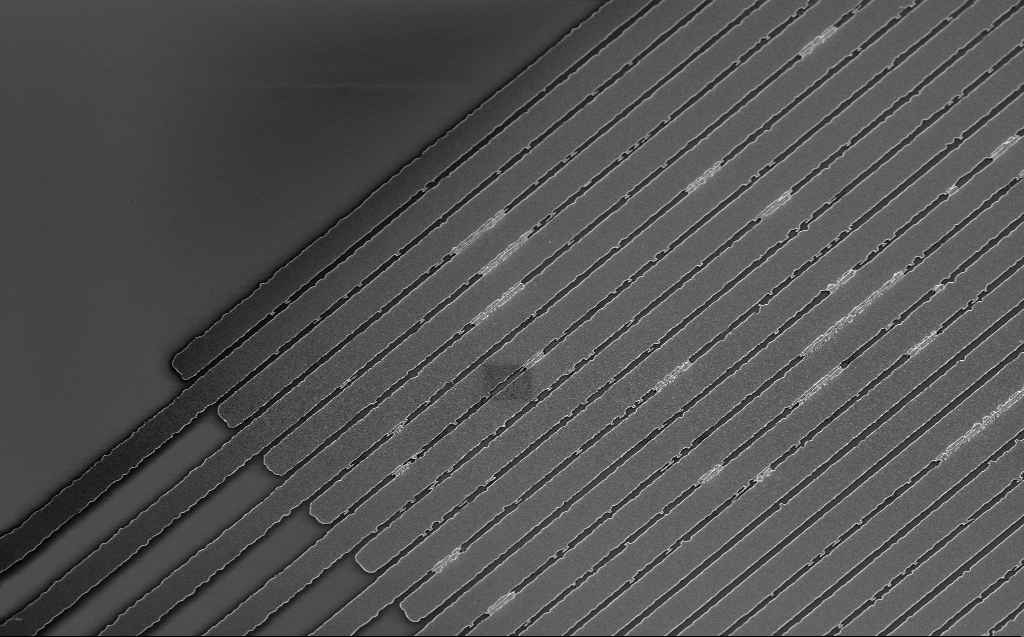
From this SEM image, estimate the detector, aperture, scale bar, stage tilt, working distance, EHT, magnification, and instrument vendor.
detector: InLens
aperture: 30 µm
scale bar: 10000 nm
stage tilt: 20°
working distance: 4 mm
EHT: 3 kV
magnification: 3.24 K X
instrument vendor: Zeiss